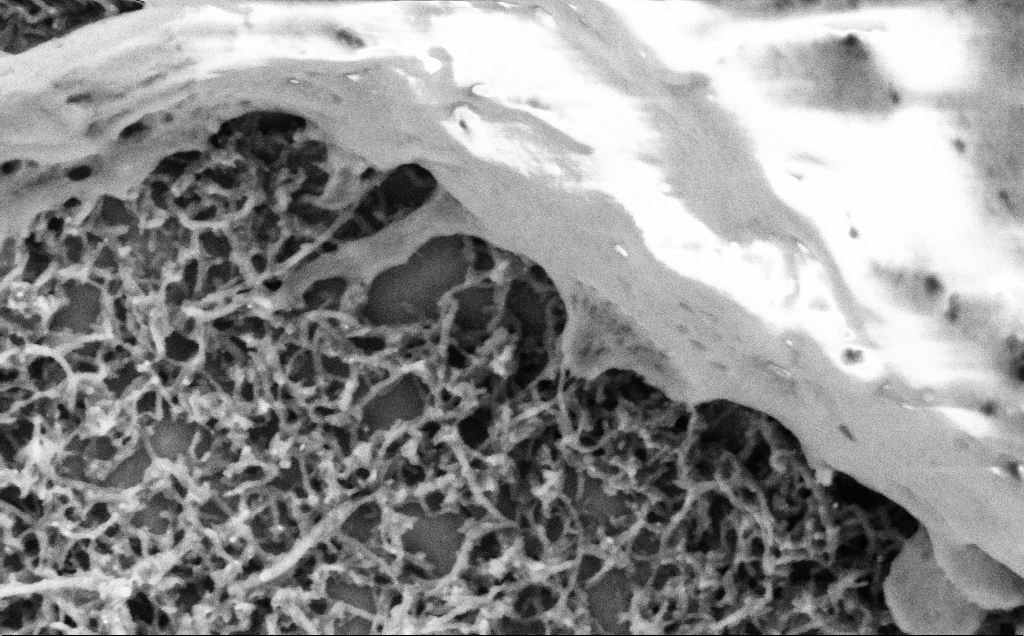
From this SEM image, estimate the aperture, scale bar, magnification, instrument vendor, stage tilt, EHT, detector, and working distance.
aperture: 30 µm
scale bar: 200 nm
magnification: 75 K X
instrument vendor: Zeiss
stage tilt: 0°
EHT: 2 kV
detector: SE2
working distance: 7.1 mm